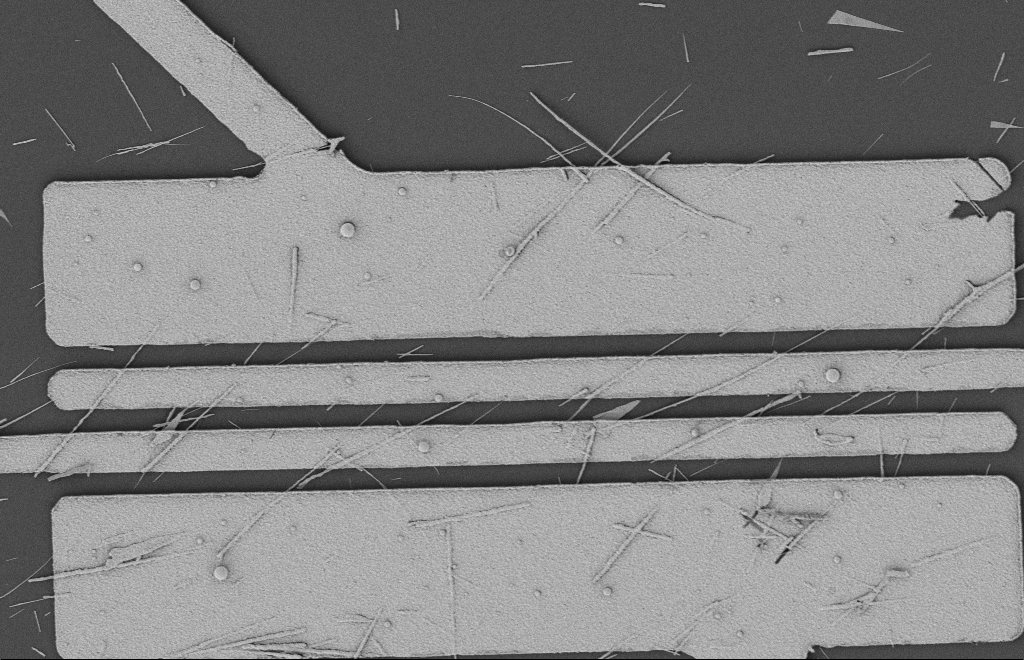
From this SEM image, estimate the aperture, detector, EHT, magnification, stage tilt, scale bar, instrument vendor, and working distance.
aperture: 20 µm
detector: SE2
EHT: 2 kV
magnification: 5.79 K X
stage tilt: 0°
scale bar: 2000 nm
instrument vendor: Zeiss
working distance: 12 mm